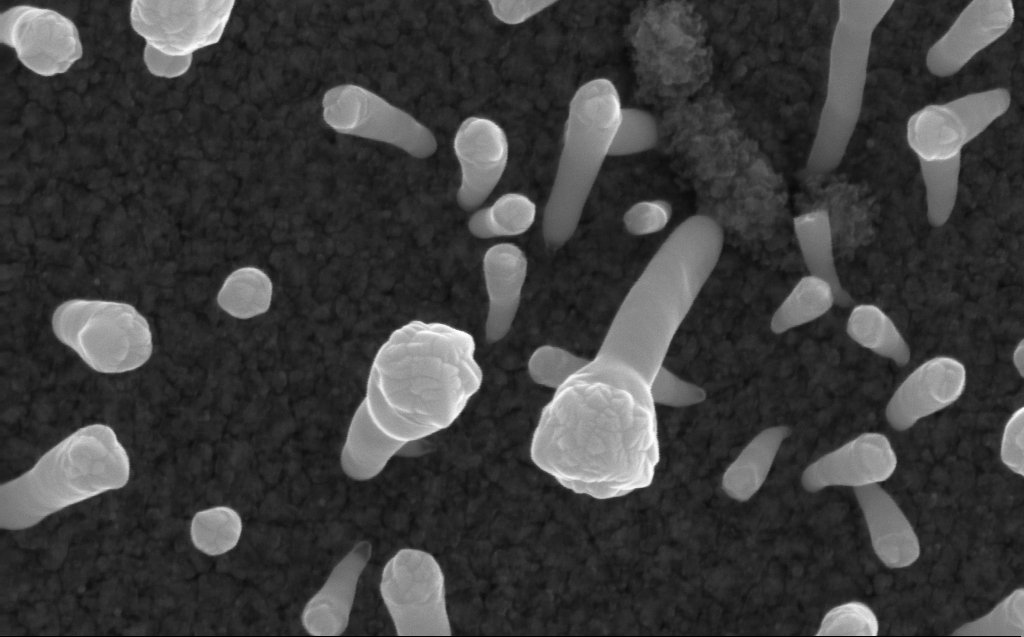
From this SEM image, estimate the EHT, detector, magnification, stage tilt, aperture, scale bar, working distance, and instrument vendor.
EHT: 10 kV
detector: InLens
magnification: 158.01 K X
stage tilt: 0°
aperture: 30 µm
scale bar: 200 nm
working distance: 3 mm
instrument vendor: Zeiss